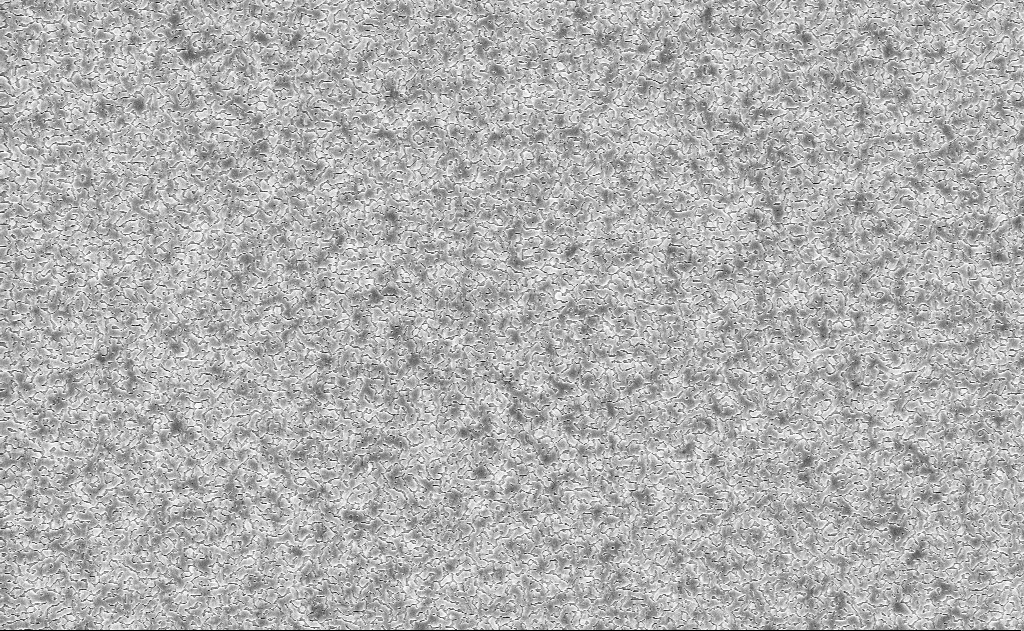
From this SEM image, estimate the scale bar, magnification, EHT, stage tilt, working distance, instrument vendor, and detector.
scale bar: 2000 nm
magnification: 10 K X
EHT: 10 kV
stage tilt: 0°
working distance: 11 mm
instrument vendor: Zeiss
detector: InLens